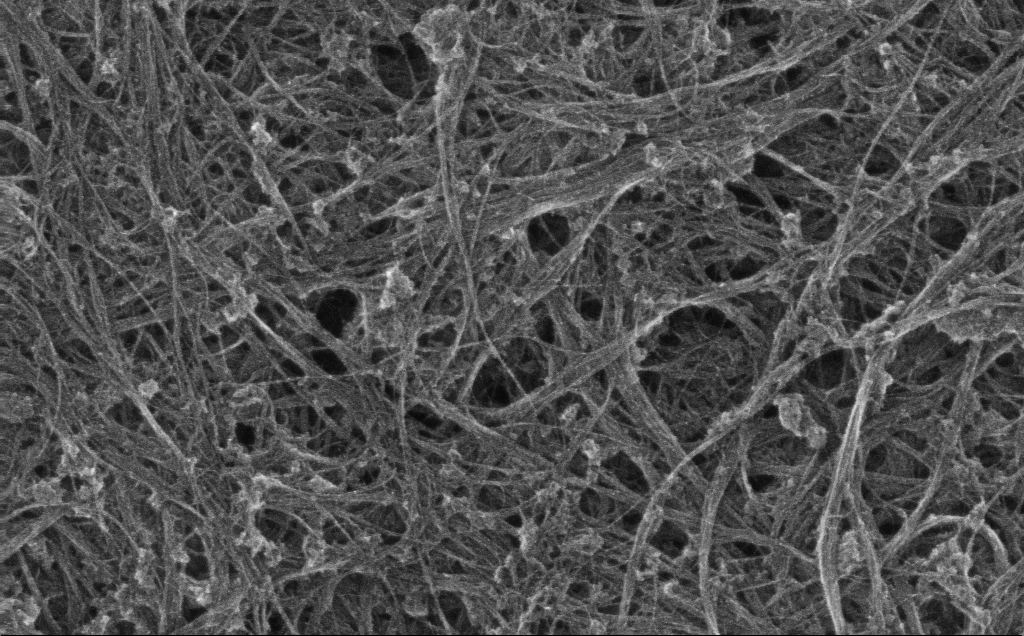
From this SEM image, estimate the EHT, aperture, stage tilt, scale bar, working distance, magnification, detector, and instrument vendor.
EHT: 10 kV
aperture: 30 µm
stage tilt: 0°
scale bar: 1000 nm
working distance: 3 mm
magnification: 67.46 K X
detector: InLens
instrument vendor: Zeiss